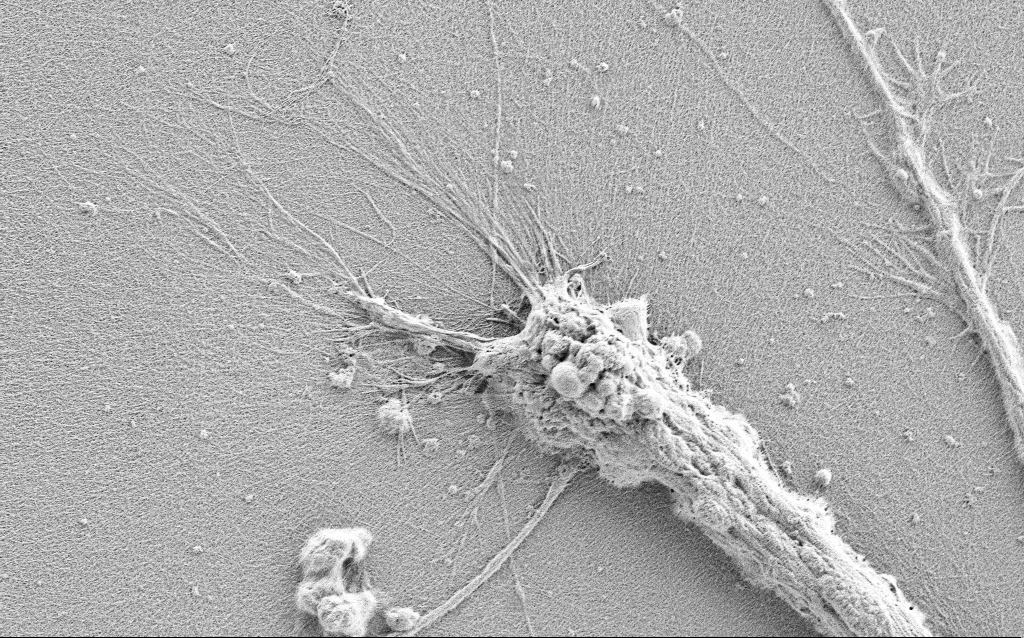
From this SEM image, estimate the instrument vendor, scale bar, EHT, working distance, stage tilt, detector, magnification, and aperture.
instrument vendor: Zeiss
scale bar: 10000 nm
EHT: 0.9 kV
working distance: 3 mm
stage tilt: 0°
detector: SE2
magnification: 5 K X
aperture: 30 µm